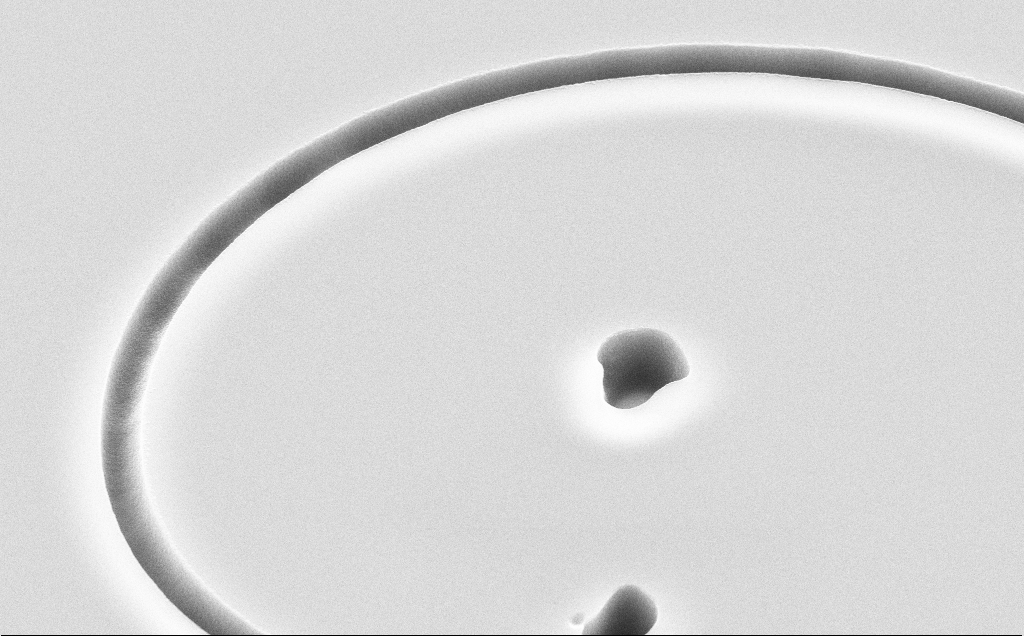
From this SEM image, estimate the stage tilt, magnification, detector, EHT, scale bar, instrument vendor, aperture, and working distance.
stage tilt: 45°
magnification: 12.37 K X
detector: SE2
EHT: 10 kV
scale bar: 2000 nm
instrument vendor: Zeiss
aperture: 30 µm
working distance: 11 mm